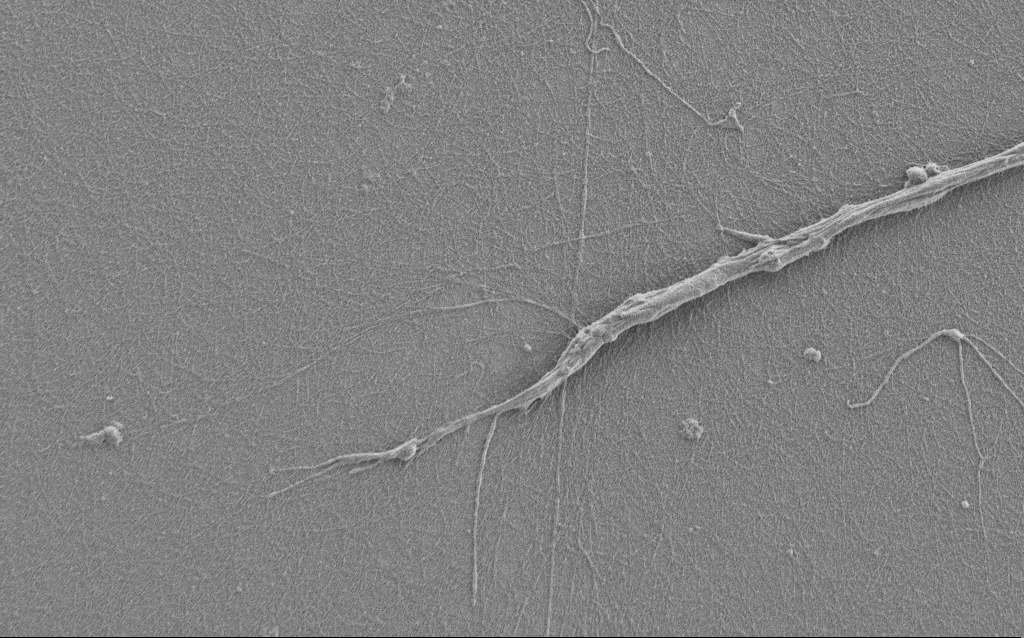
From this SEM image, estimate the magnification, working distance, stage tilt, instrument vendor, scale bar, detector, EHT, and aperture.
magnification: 7.5 K X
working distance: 6 mm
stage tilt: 0°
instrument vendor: Zeiss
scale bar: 2000 nm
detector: SE2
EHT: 1 kV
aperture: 30 µm